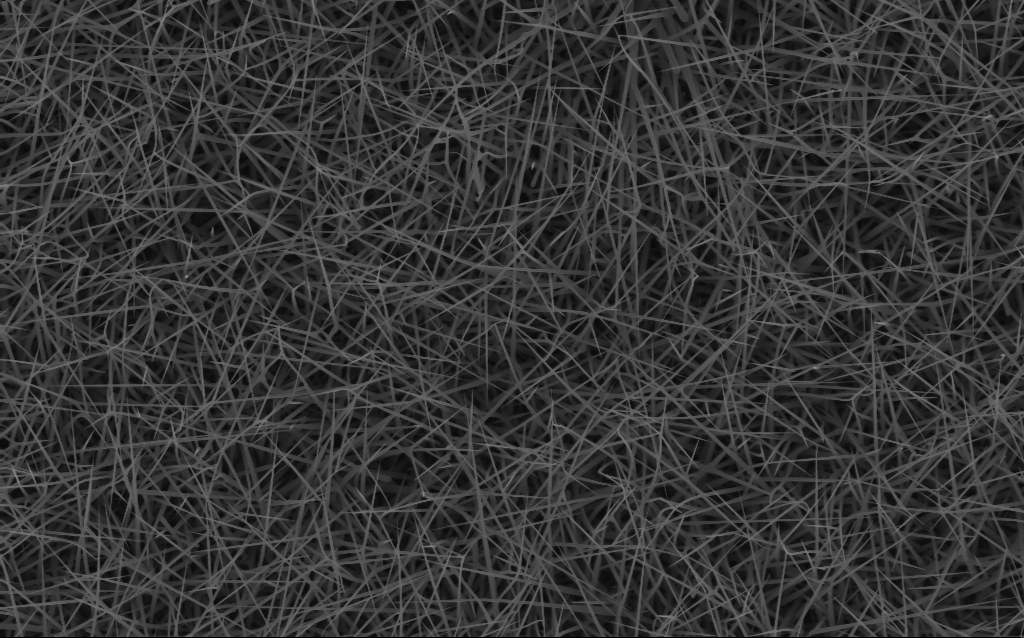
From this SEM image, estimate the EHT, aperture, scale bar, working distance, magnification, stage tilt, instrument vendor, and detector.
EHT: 10 kV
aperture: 30 µm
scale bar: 2000 nm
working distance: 6 mm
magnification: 10 K X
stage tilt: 0°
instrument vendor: Zeiss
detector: InLens